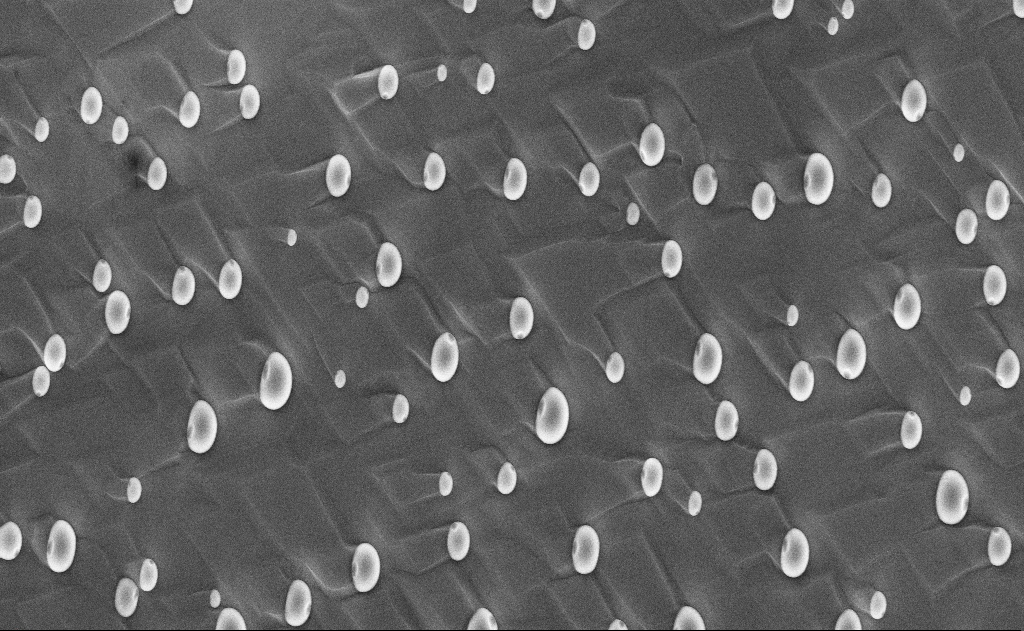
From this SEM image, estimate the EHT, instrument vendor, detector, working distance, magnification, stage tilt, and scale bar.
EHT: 10 kV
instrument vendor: Zeiss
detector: InLens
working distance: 15 mm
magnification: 10 K X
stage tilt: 0°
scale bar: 2000 nm